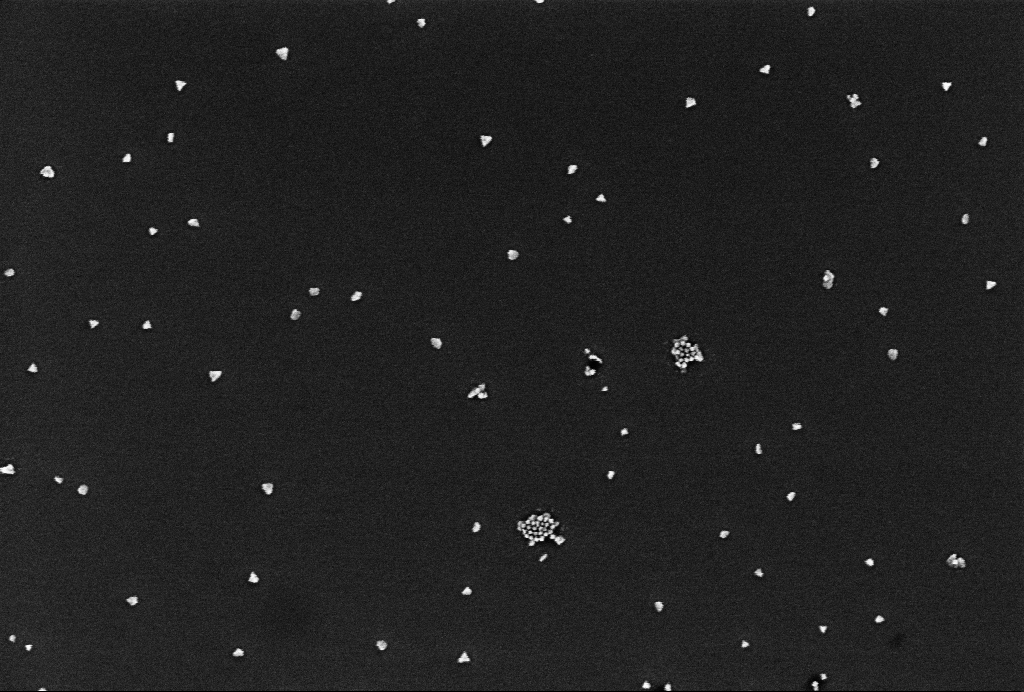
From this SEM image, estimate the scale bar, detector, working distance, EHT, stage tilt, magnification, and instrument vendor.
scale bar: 200 nm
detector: InLens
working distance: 3.4 mm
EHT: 1 kV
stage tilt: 0°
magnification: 86.59 K X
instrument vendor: Zeiss